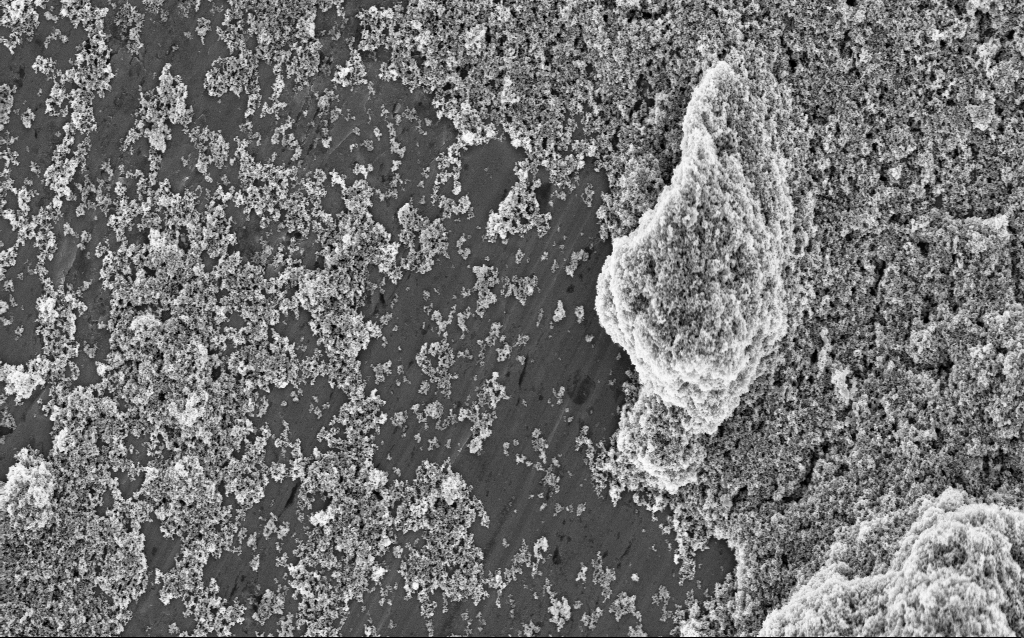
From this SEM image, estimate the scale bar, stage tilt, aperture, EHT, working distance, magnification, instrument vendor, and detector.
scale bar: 1000 nm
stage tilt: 0°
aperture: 30 µm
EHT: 5 kV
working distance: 9.6 mm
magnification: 14.66 K X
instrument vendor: Zeiss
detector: InLens